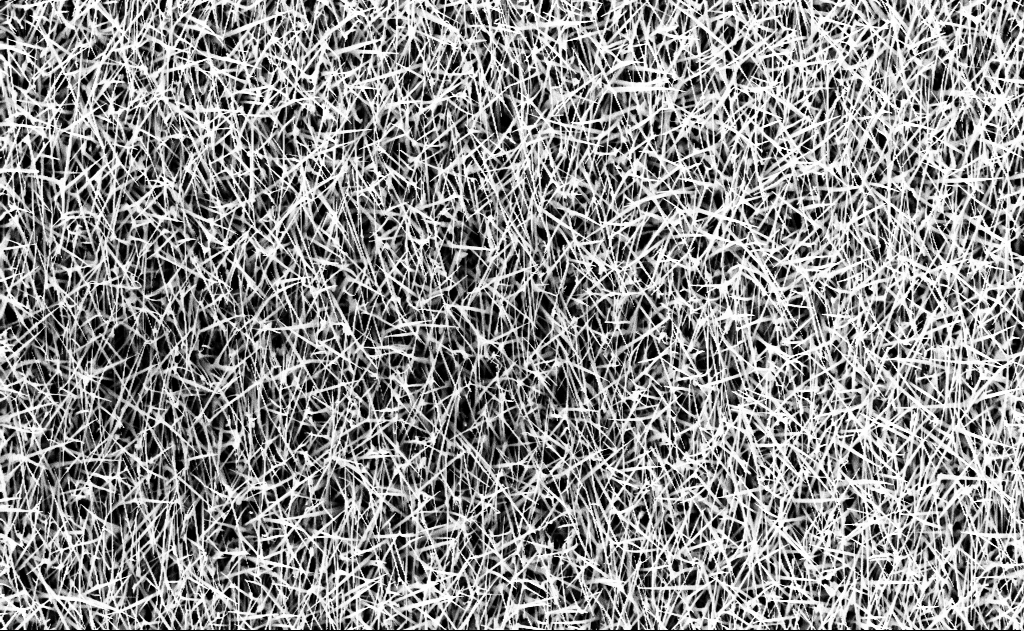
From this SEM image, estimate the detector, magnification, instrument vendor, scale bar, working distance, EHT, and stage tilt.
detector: InLens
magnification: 10 K X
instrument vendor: Zeiss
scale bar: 2000 nm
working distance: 16 mm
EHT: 10 kV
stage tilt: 0°